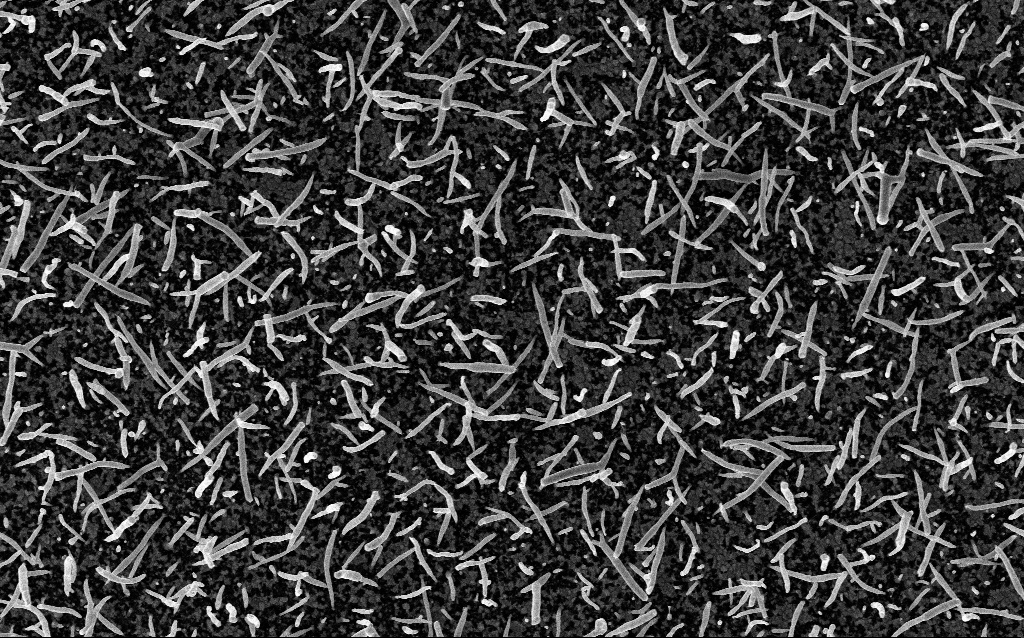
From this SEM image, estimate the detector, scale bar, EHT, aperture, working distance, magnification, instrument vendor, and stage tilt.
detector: InLens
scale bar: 1000 nm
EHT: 5 kV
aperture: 30 µm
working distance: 2.6 mm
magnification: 20 K X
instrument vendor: Zeiss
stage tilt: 0°